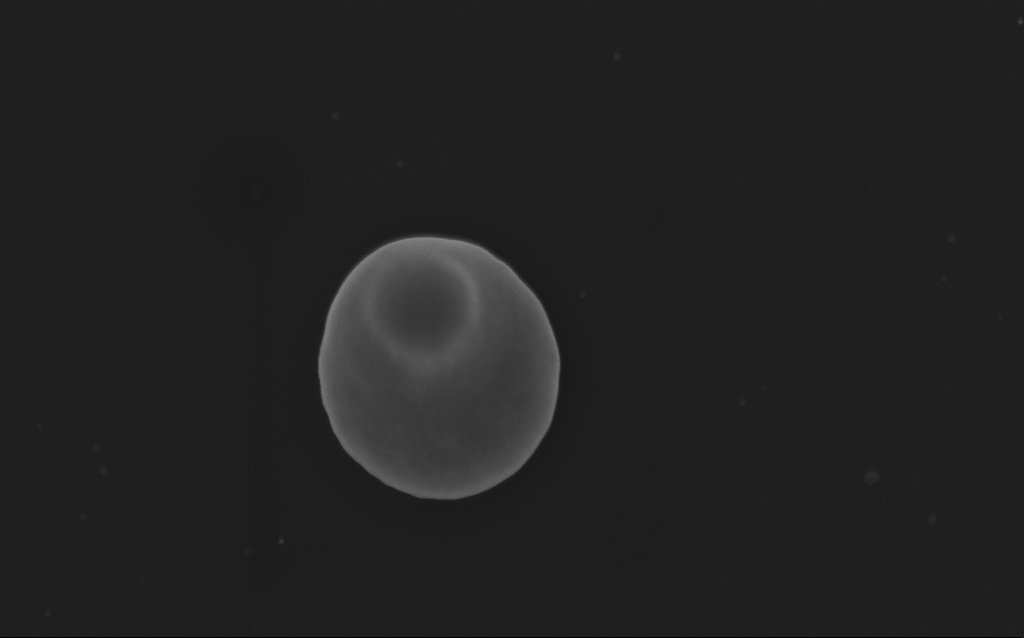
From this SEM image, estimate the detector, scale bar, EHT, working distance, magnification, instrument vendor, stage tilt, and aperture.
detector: InLens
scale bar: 200 nm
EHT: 5 kV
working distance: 4 mm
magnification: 91.05 K X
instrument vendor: Zeiss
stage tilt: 0°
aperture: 30 µm